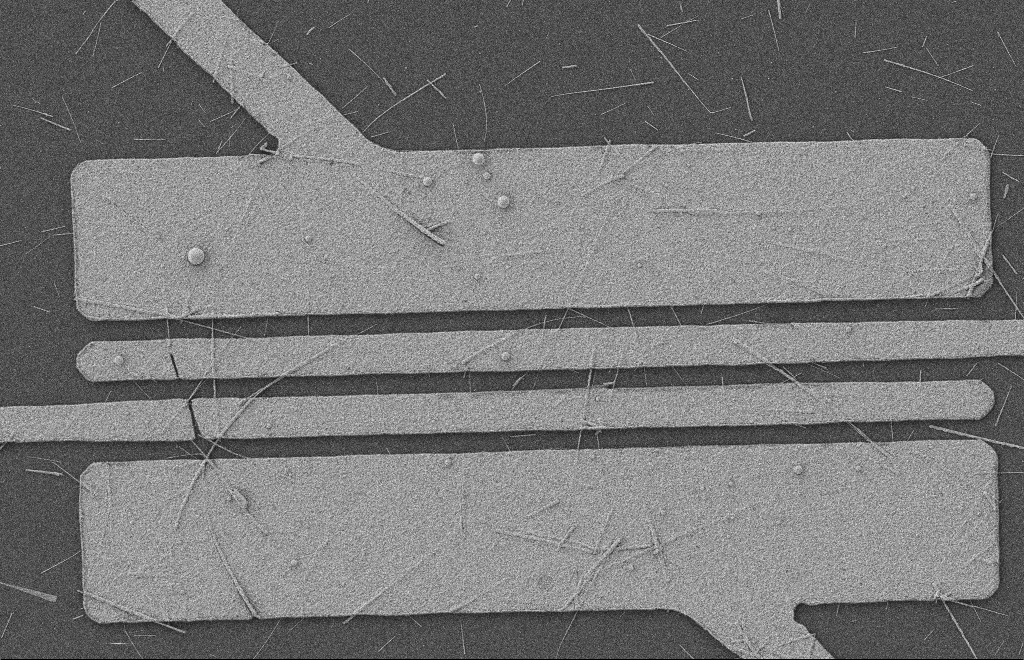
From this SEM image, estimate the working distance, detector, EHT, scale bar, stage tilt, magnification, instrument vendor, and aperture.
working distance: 8 mm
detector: SE2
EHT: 2 kV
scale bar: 2000 nm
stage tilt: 0°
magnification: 5.54 K X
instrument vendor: Zeiss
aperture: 20 µm